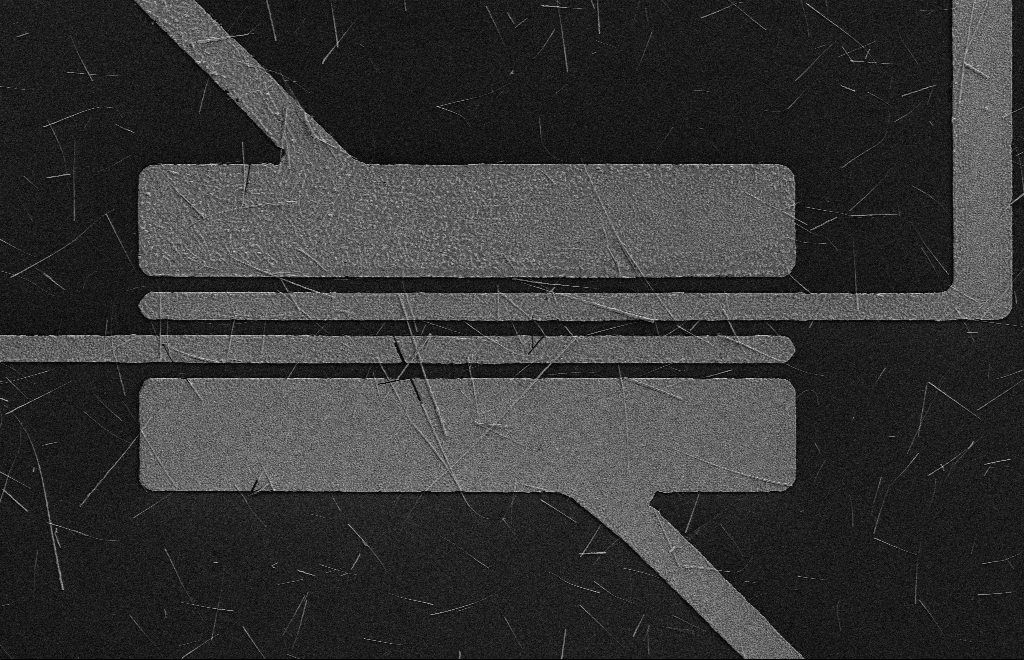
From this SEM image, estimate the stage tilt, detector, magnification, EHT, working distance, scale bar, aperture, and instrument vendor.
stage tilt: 0°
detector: SE2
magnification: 3.97 K X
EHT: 5 kV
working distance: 16 mm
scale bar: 10000 nm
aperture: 10 µm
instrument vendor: Zeiss